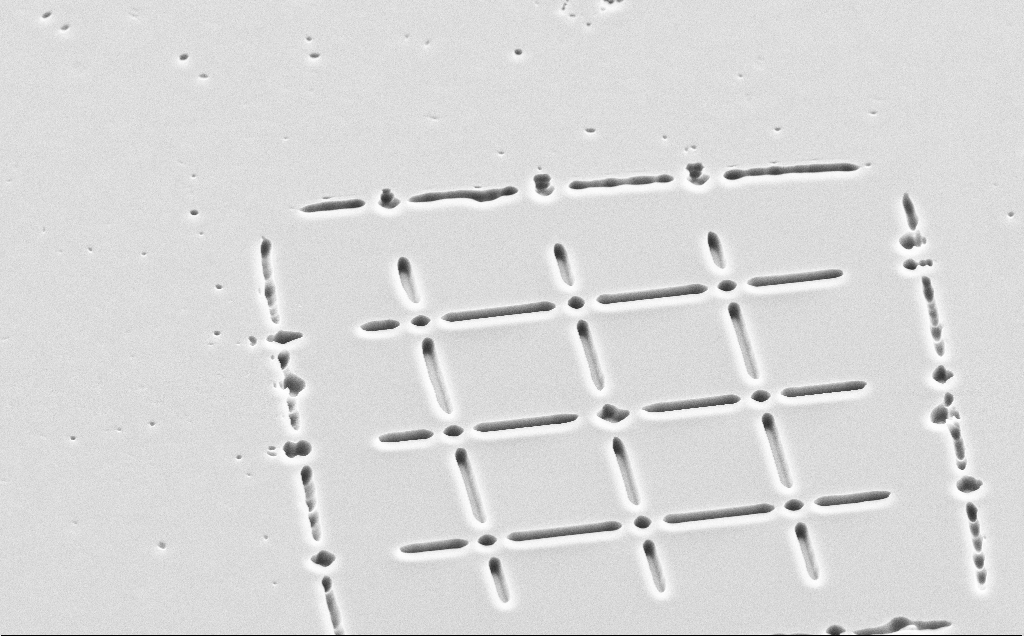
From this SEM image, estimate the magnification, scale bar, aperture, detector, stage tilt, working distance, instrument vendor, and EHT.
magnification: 2.85 K X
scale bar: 10000 nm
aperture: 30 µm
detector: SE2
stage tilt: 45°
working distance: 11 mm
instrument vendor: Zeiss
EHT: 10 kV